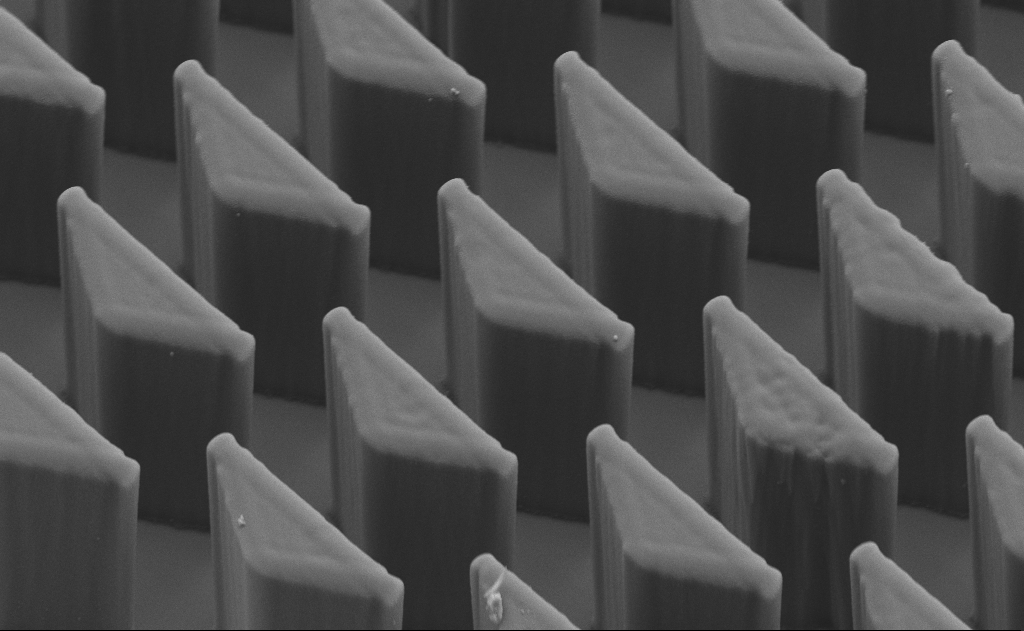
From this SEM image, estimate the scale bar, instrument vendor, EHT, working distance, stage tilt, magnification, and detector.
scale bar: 20000 nm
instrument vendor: Zeiss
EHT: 10 kV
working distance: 11 mm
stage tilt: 45°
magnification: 2.63 K X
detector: SE2